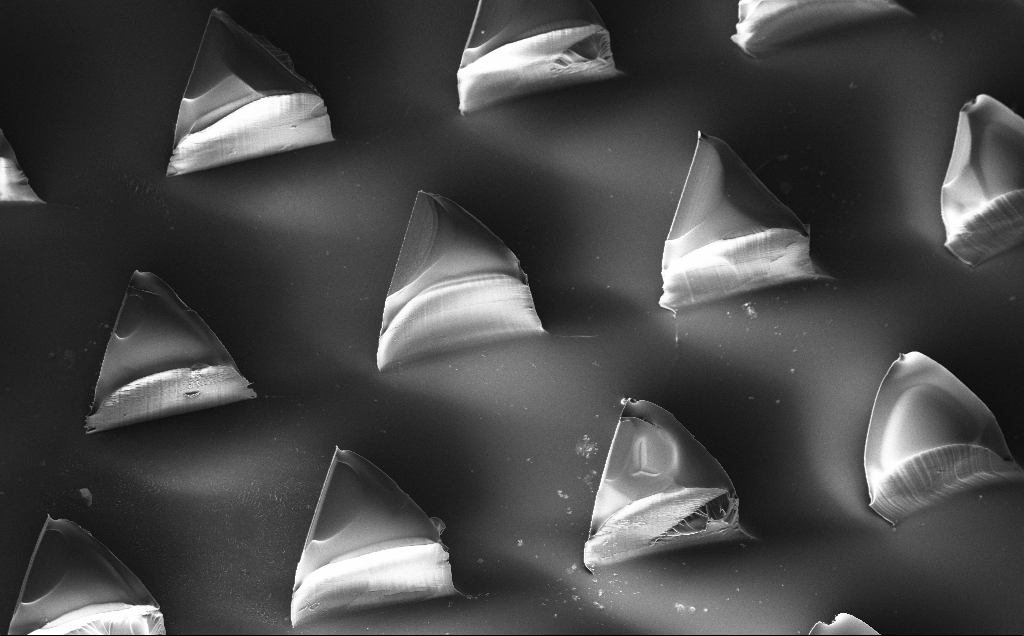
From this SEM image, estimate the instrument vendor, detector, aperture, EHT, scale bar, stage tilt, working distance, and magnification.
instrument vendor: Zeiss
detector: InLens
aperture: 30 µm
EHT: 10 kV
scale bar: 100000 nm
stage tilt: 20°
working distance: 9 mm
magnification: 0.239 K X